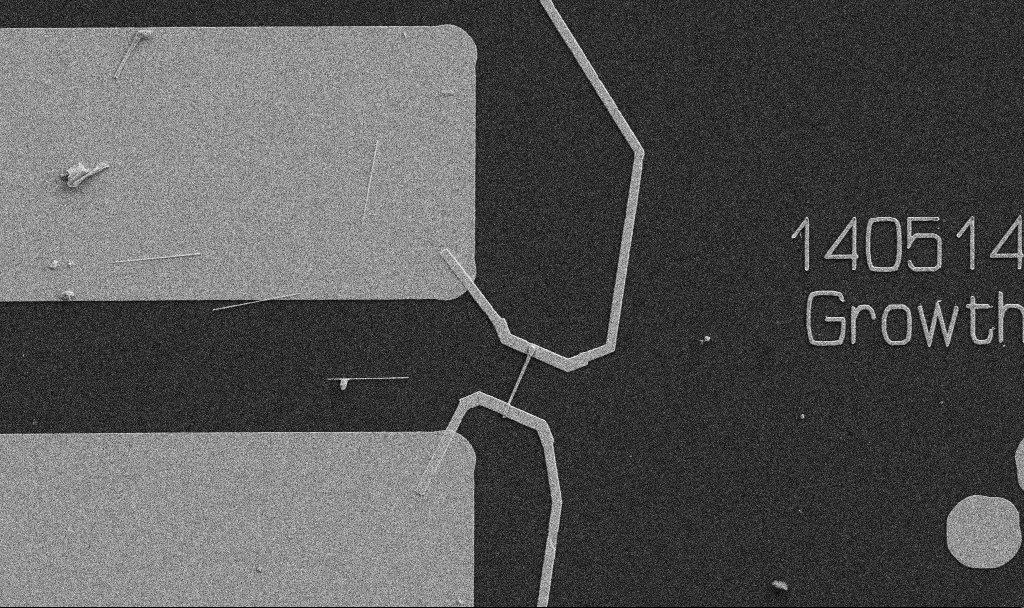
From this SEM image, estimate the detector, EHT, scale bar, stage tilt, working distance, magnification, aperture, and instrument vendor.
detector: SE2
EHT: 5 kV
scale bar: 10000 nm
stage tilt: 0°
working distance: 10.7 mm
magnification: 5 K X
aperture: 30 µm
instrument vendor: Zeiss